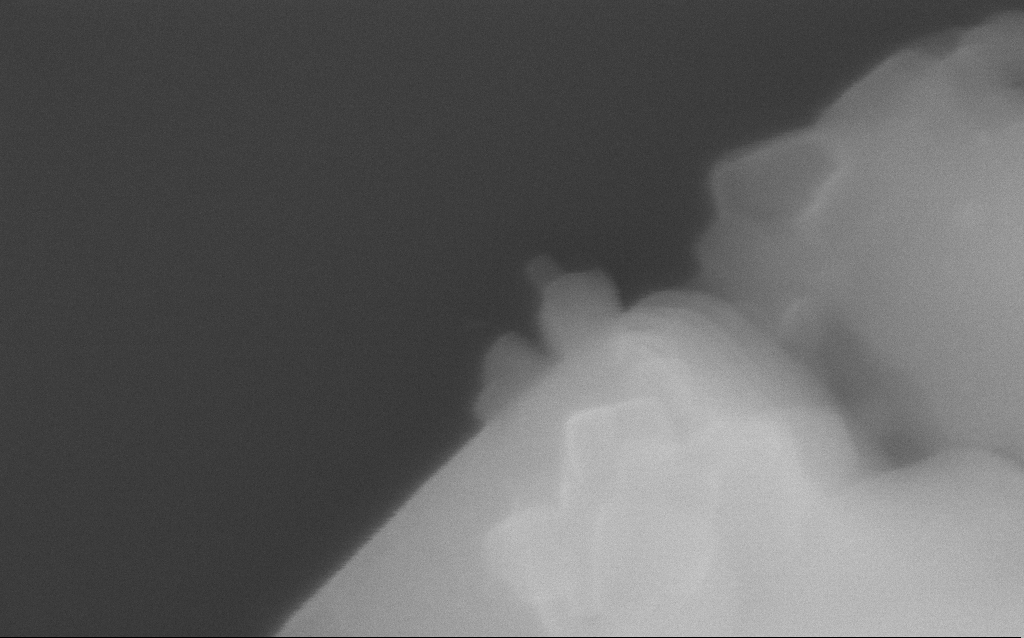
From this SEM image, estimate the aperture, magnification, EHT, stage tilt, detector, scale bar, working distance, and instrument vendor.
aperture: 30 µm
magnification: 380.96 K X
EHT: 10 kV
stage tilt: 0°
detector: InLens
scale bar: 100 nm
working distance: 3 mm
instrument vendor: Zeiss